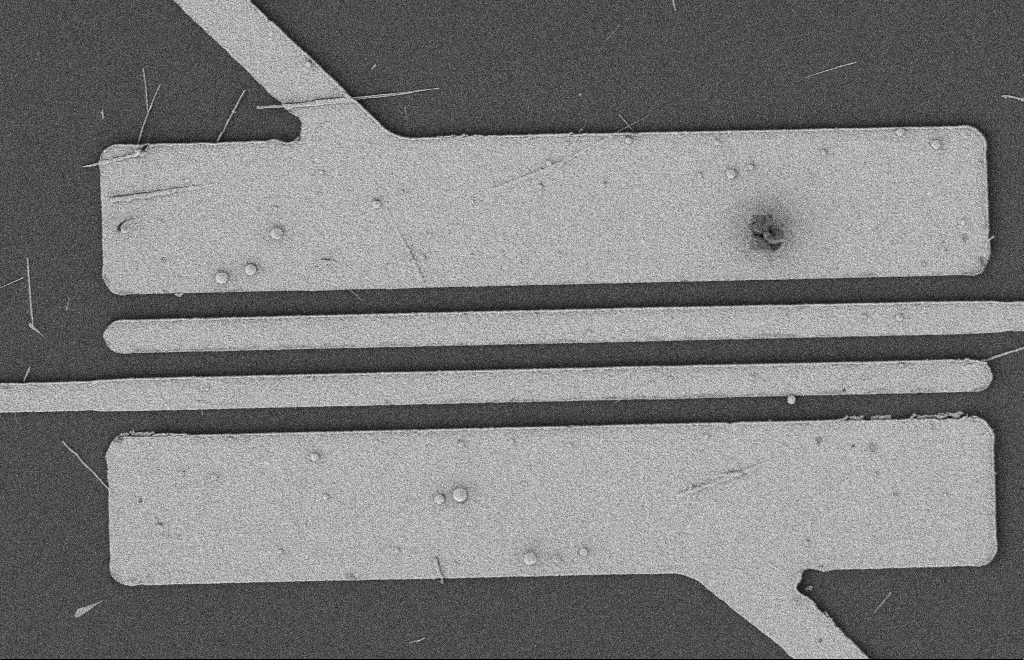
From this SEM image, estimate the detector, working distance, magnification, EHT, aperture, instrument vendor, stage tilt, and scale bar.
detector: SE2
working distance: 12 mm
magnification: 5.31 K X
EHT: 2 kV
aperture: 20 µm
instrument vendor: Zeiss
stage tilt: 0°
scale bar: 2000 nm